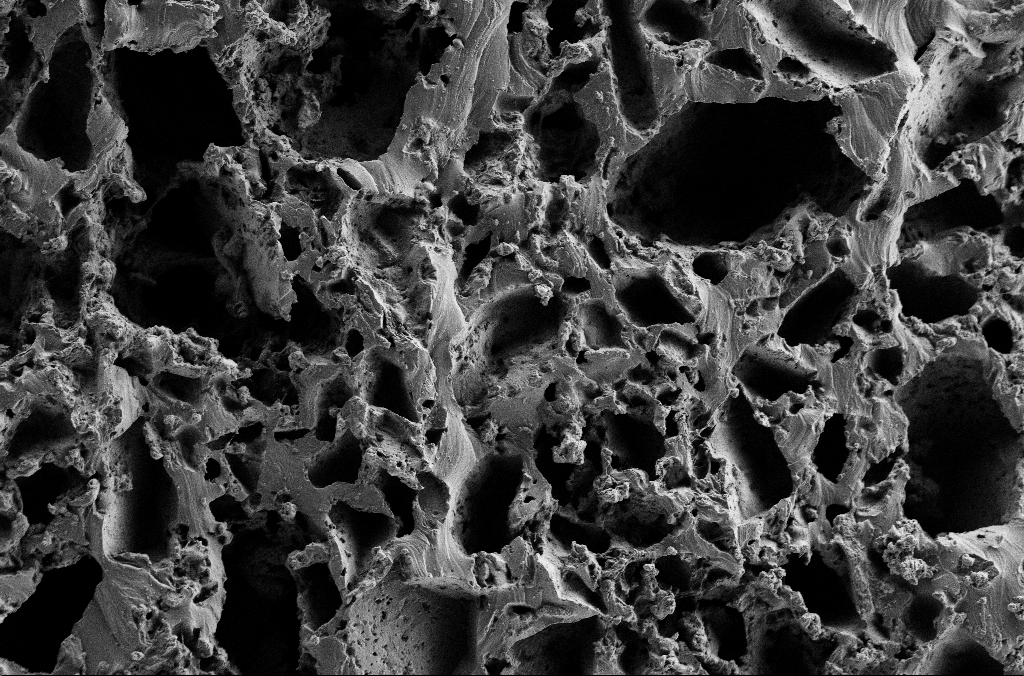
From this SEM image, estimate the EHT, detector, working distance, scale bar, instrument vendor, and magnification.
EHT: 2 kV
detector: SE2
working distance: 3 mm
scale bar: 100000 nm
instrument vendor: Zeiss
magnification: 0.5 K X